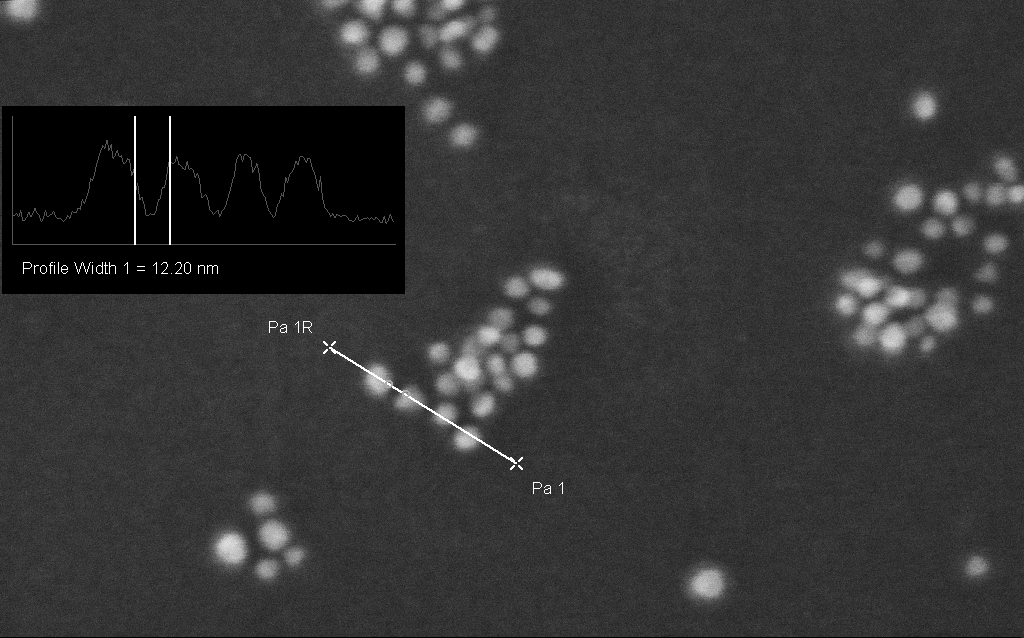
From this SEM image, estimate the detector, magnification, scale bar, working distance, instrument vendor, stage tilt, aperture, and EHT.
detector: InLens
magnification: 602.1 K X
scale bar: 100 nm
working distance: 1.8 mm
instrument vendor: Zeiss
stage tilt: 0°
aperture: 30 µm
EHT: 10 kV